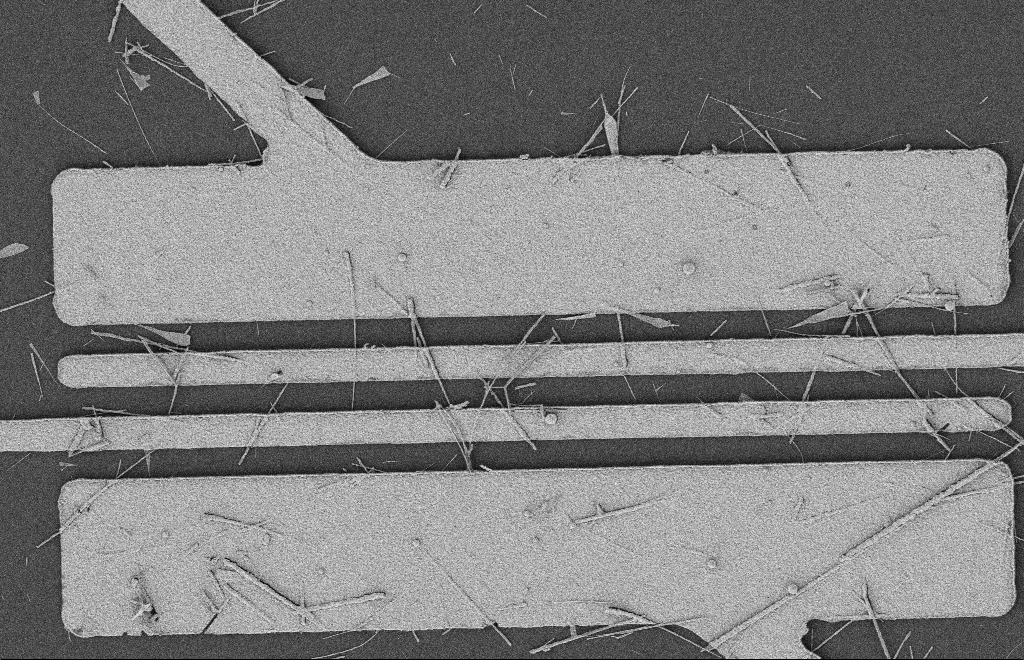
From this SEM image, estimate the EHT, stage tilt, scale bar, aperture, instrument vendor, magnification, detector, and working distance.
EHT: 2 kV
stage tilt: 0°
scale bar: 2000 nm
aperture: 20 µm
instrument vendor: Zeiss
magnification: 5.71 K X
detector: SE2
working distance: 12 mm